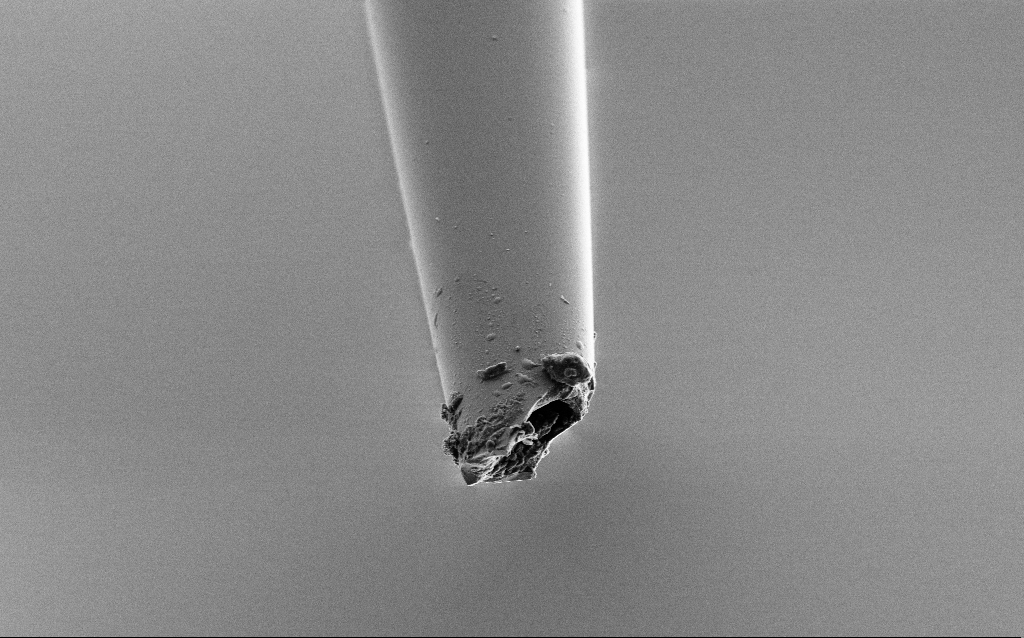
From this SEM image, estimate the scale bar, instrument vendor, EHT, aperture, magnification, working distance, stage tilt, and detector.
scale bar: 10000 nm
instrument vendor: Zeiss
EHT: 2 kV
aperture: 30 µm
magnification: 5 K X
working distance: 6 mm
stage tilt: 45°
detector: SE2